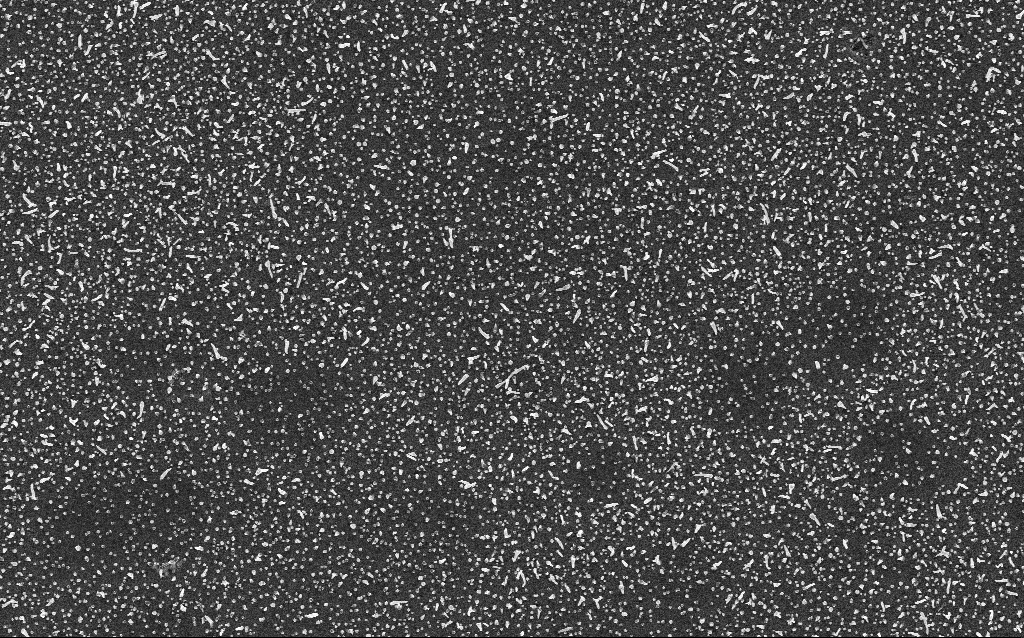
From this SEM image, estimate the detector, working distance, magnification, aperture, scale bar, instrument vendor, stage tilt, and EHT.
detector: InLens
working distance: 2 mm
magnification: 10 K X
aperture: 30 µm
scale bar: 2000 nm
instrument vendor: Zeiss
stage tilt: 0°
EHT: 5 kV